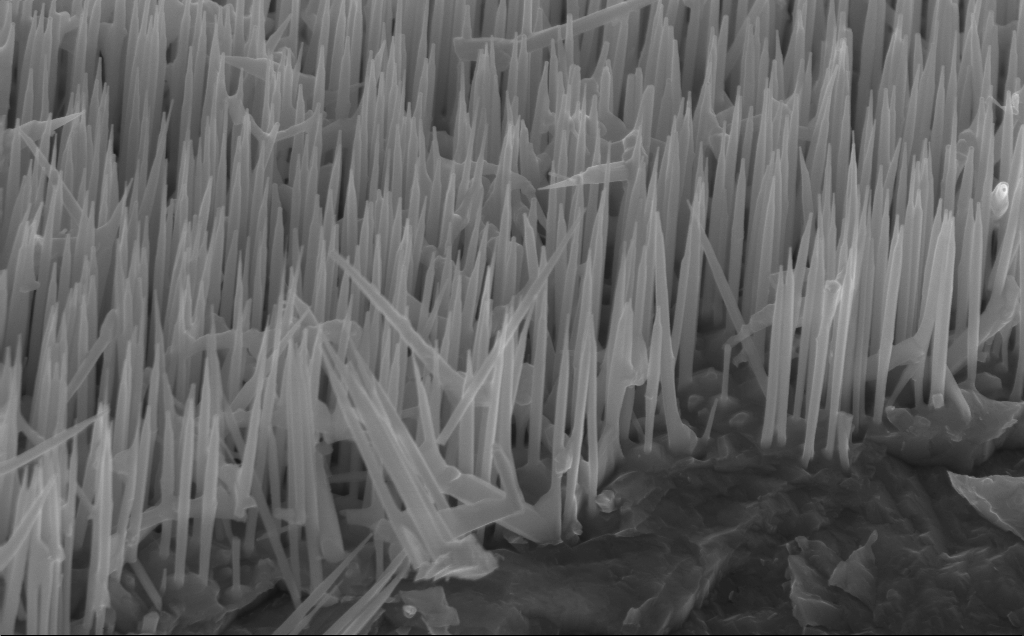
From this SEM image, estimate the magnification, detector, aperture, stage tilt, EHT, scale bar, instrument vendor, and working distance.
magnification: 40 K X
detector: InLens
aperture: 30 µm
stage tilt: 45°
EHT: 12 kV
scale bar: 1000 nm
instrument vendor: Zeiss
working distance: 5 mm